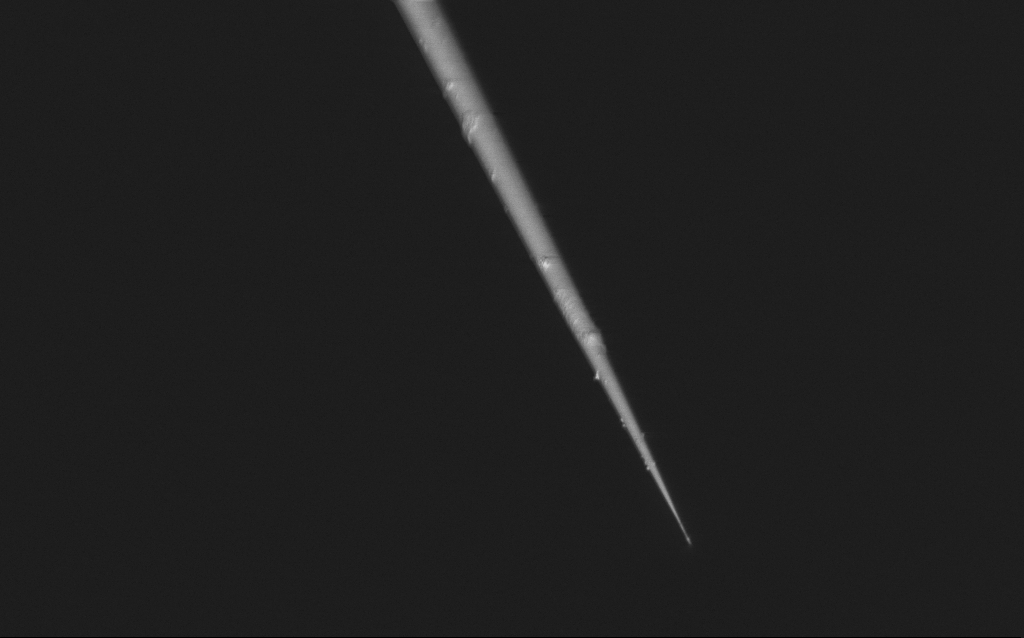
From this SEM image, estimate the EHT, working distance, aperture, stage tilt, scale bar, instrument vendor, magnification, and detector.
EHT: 1 kV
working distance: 7 mm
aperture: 30 µm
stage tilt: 45°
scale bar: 2000 nm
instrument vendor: Zeiss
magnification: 10 K X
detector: InLens